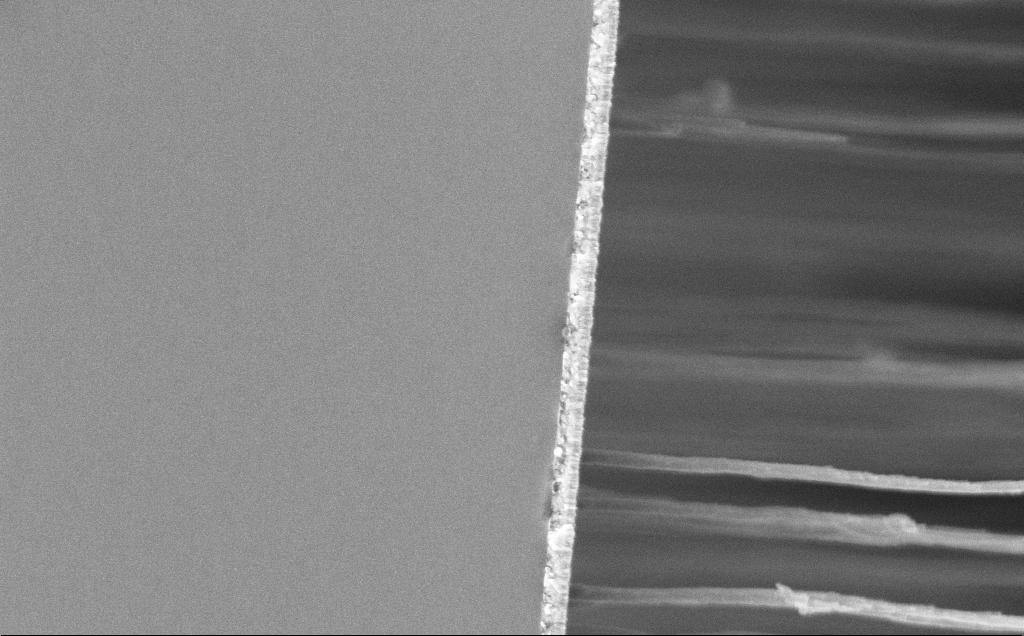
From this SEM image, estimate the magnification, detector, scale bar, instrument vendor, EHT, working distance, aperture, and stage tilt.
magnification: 39.12 K X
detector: InLens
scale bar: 1000 nm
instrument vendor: Zeiss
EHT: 5 kV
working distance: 12 mm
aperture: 30 µm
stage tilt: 0°